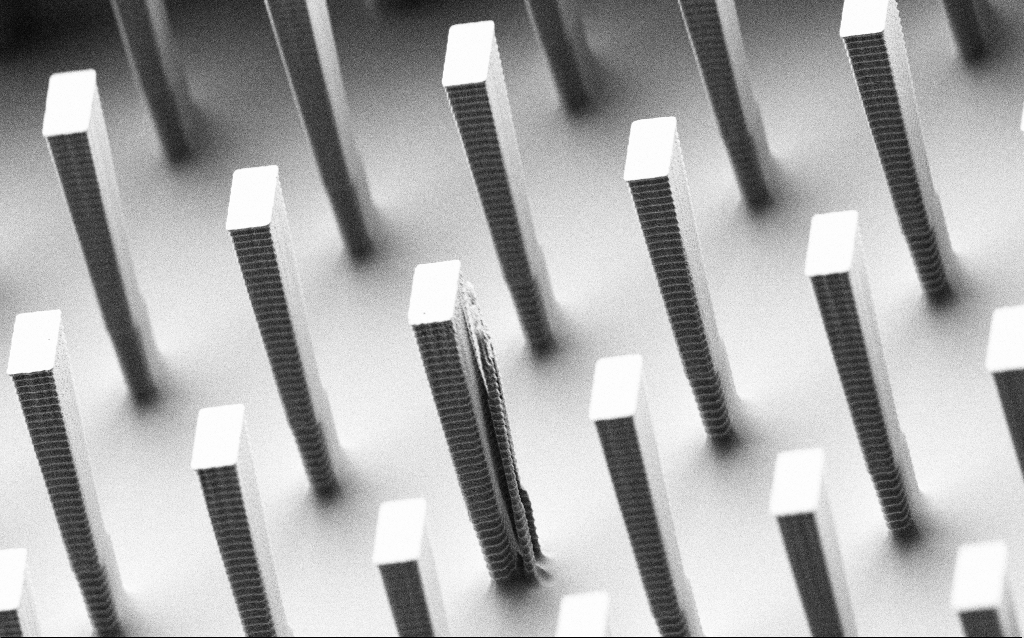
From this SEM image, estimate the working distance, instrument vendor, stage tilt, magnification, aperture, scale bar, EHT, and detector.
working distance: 3.2 mm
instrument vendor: Zeiss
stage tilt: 50°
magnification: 8.53 K X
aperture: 30 µm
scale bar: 2000 nm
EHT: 1.5 kV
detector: SE2